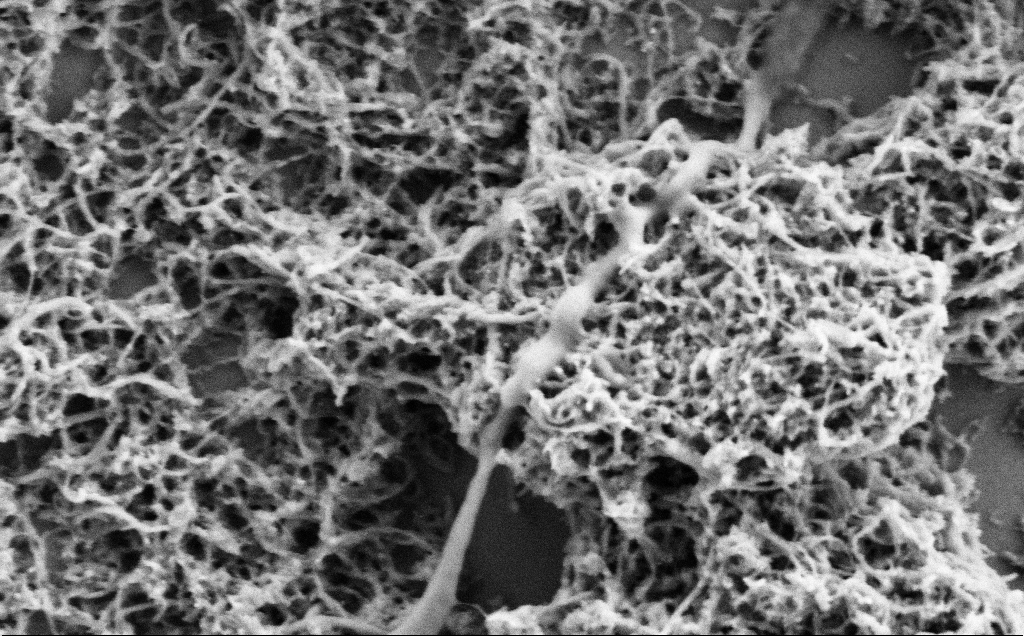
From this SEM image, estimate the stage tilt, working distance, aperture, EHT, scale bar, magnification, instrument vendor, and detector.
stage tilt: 0°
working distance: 7.1 mm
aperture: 30 µm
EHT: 2 kV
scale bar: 1000 nm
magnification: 60 K X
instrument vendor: Zeiss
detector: SE2